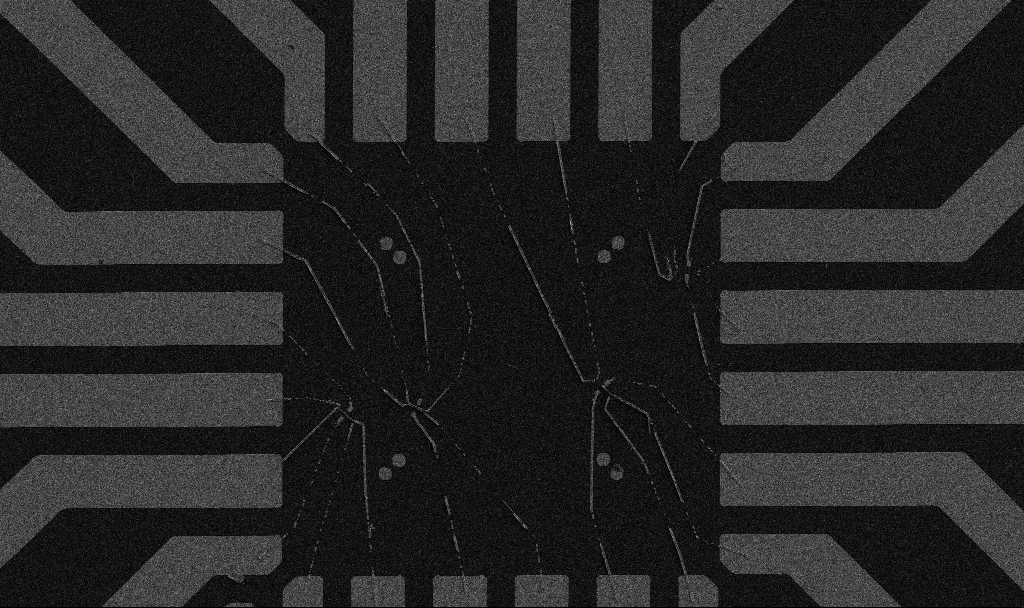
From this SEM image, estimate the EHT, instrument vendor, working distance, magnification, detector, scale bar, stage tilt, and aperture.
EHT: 5 kV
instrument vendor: Zeiss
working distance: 10.7 mm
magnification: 1 K X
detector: SE2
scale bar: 20000 nm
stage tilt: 0°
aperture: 30 µm